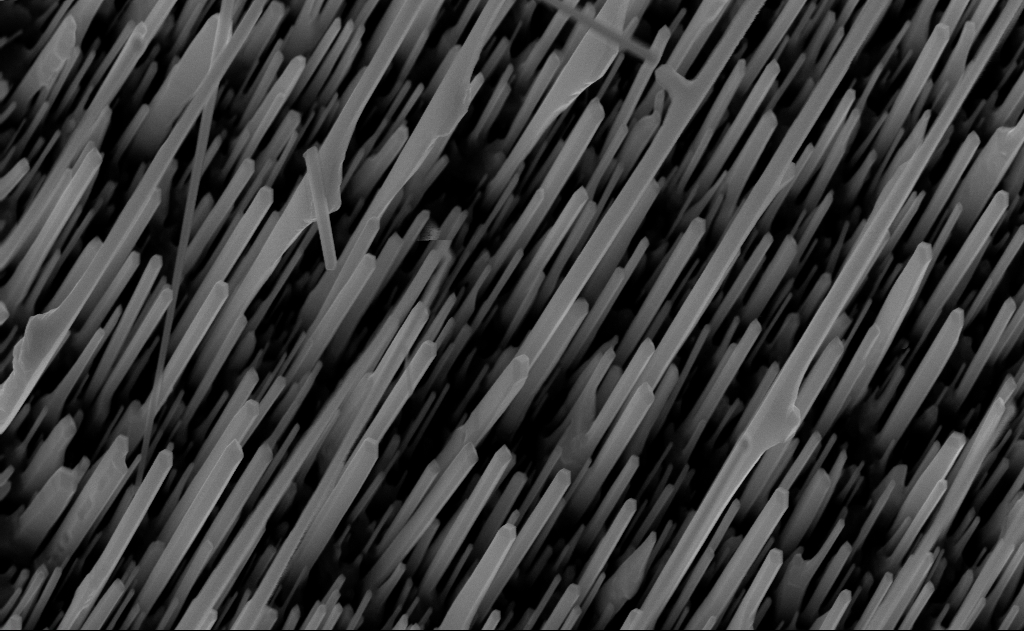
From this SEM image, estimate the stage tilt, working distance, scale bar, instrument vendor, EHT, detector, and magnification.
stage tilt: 0°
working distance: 5 mm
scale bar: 1000 nm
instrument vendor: Zeiss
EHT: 10 kV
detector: InLens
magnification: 40 K X